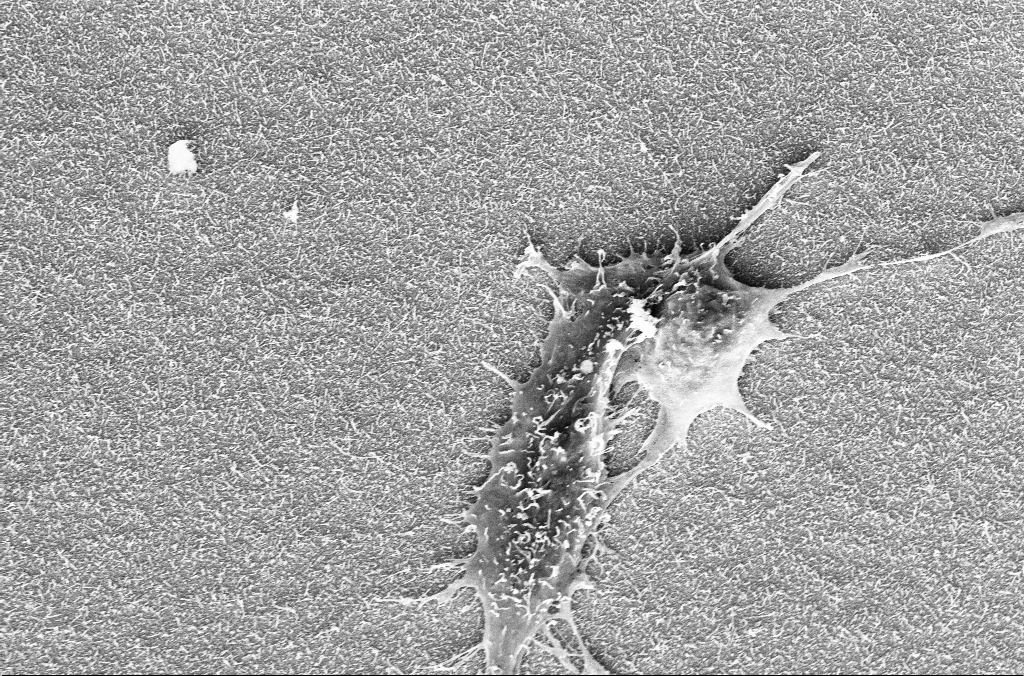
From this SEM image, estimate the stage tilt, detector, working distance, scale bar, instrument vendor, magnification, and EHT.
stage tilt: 17.3°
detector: InLens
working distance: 8.1 mm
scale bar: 10000 nm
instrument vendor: Zeiss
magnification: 5.43 K X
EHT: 10 kV